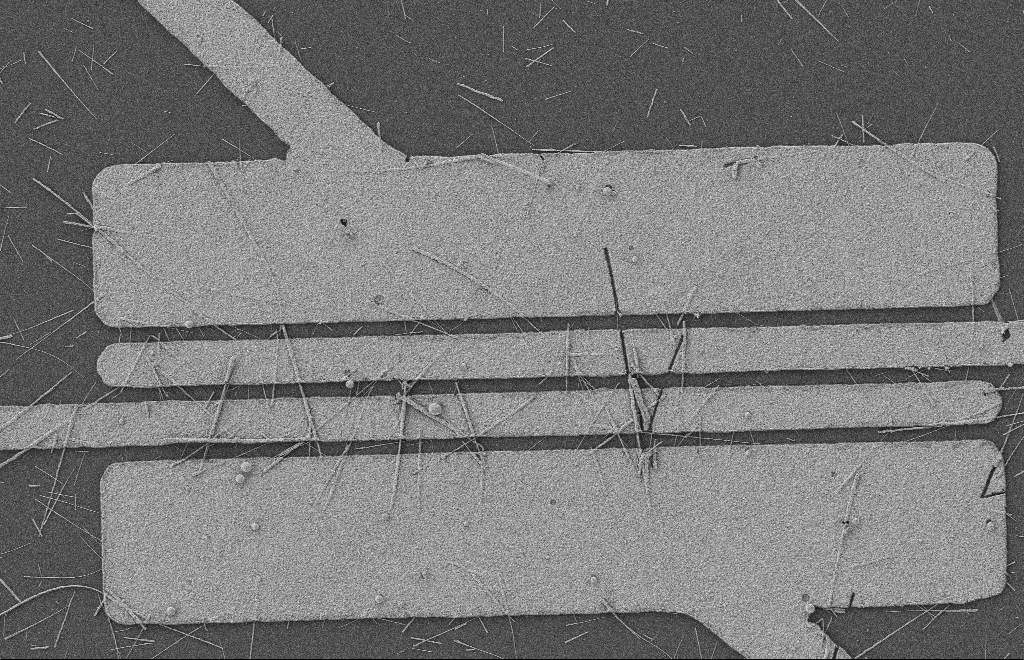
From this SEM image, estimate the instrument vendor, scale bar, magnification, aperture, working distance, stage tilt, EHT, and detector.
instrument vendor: Zeiss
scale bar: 2000 nm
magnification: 5.42 K X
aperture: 20 µm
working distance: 8 mm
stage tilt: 0°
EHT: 2 kV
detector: SE2